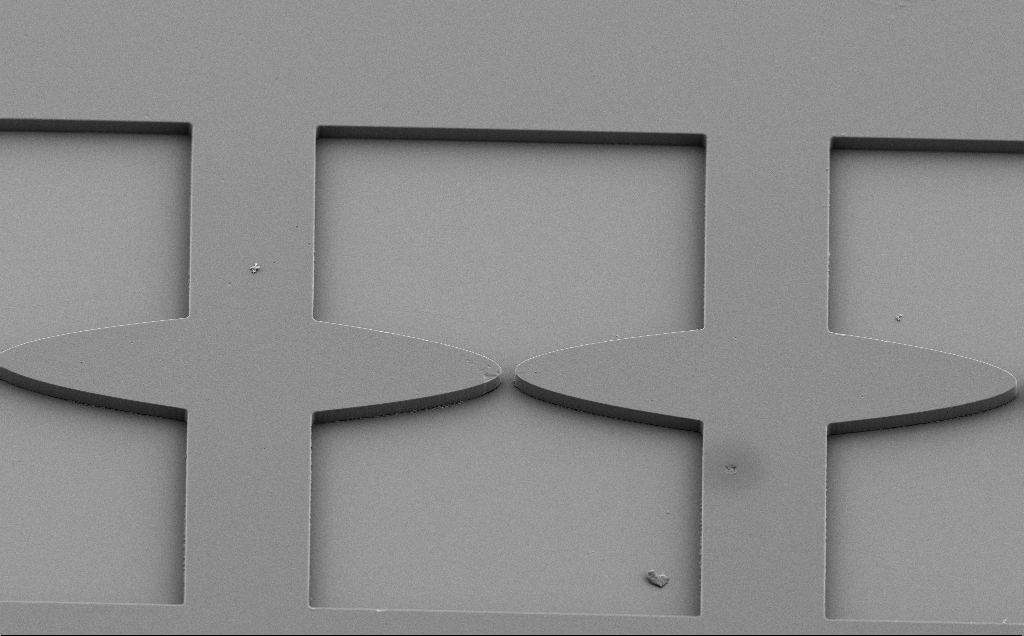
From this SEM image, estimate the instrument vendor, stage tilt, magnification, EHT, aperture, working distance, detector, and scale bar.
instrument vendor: Zeiss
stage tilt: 40°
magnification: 0.93 K X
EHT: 5 kV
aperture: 30 µm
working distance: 8 mm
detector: SE2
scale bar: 20000 nm